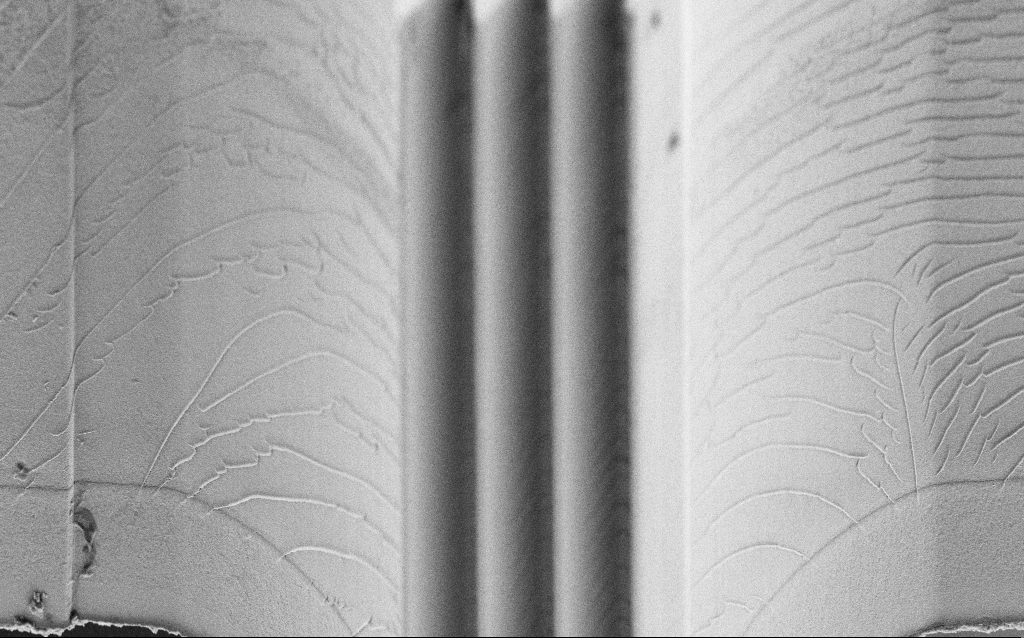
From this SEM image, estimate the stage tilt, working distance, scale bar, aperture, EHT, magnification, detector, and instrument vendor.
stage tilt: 45°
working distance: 7 mm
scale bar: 10000 nm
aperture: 30 µm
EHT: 5 kV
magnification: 2.51 K X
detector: SE2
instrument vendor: Zeiss